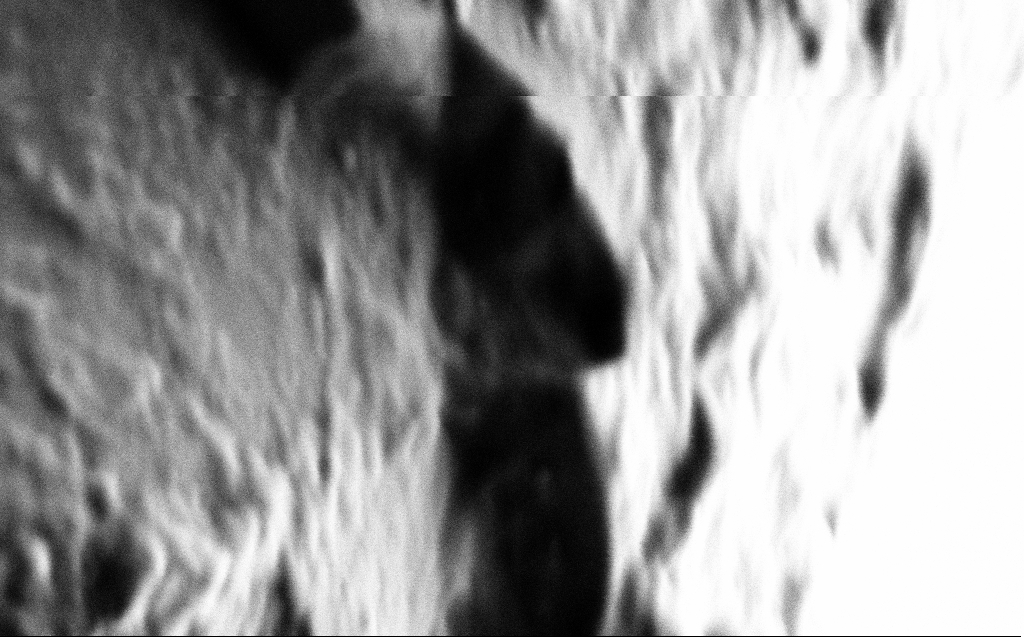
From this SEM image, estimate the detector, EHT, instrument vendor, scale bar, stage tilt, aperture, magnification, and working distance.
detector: SE2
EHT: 1 kV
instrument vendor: Zeiss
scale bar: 200 nm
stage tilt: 45°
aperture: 30 µm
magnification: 179.68 K X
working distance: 4 mm